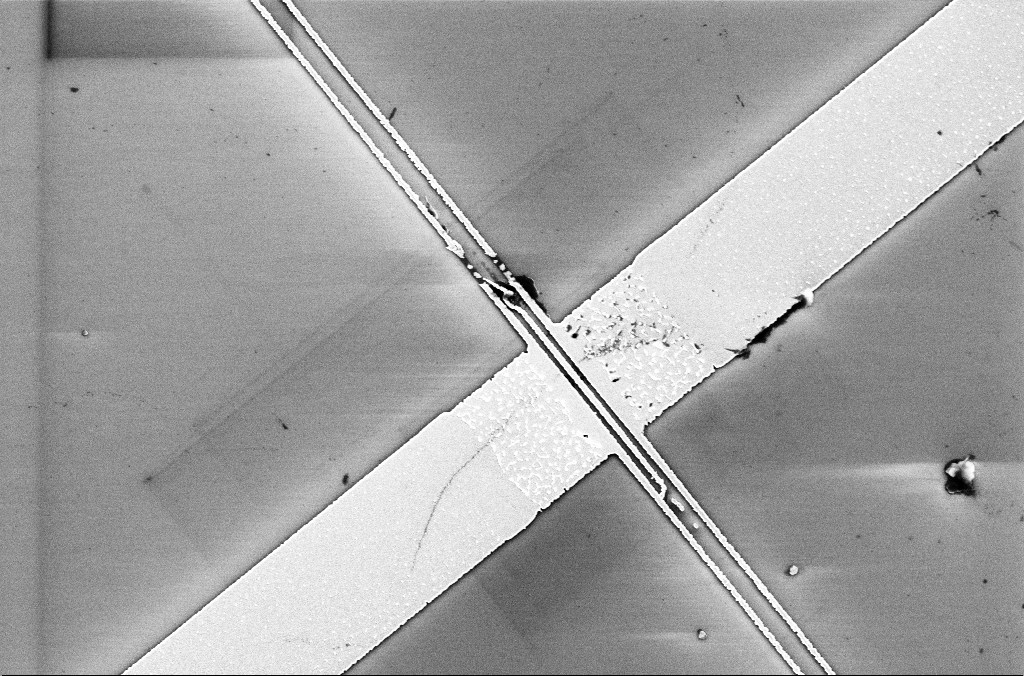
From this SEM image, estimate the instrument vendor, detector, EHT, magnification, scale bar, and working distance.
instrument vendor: Zeiss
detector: InLens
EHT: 5 kV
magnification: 10.15 K X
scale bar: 2000 nm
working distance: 3.3 mm